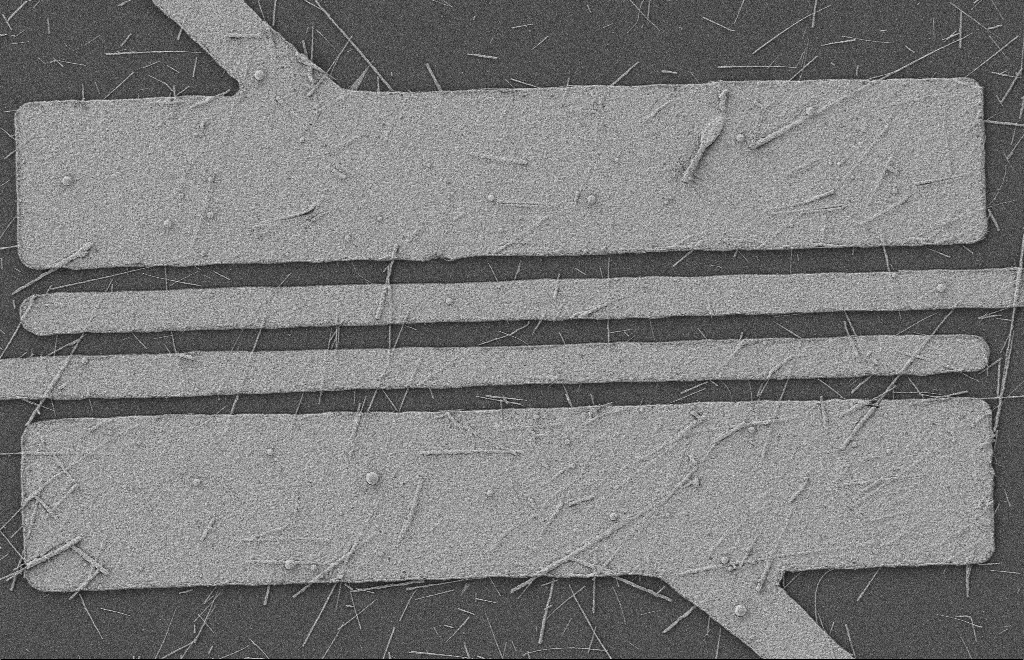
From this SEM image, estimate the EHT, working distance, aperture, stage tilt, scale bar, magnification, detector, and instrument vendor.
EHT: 2 kV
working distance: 8 mm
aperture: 20 µm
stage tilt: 0°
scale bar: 2000 nm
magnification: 5.85 K X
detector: SE2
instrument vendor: Zeiss